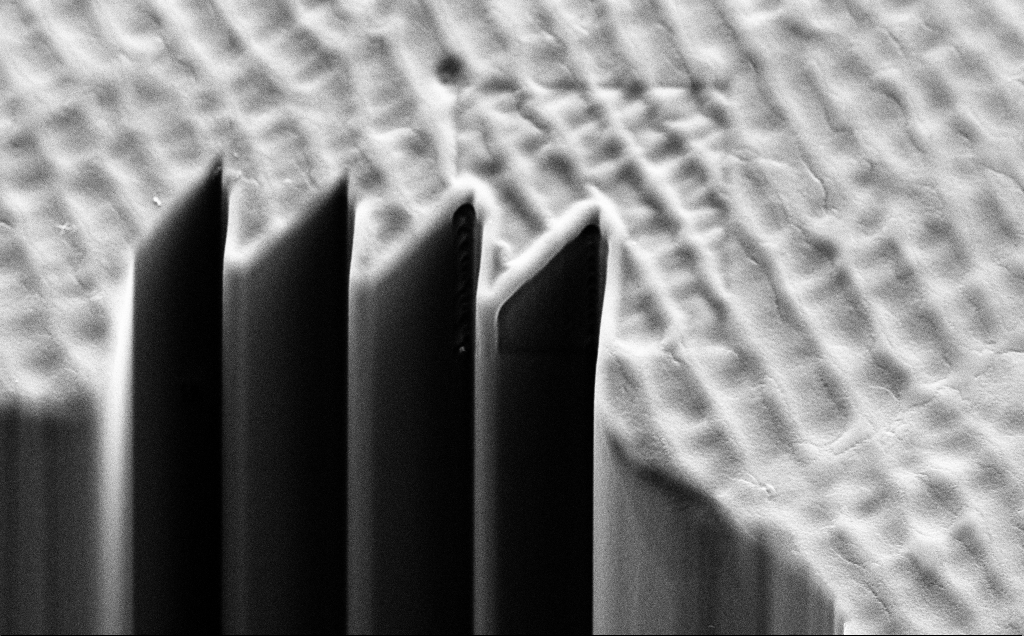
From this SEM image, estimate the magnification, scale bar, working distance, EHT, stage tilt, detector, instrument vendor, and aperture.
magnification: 4.06 K X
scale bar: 10000 nm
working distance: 11 mm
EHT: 10 kV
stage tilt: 45°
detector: SE2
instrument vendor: Zeiss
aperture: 30 µm